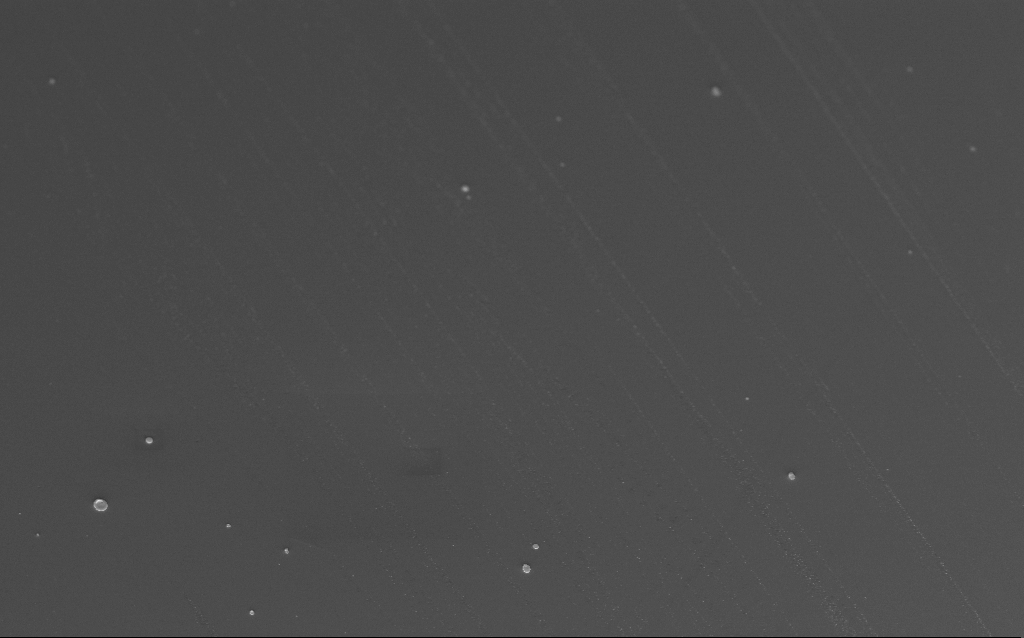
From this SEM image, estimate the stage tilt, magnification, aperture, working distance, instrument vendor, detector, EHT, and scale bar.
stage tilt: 40°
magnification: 2.99 K X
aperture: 30 µm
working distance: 5 mm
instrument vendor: Zeiss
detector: InLens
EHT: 3 kV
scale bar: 10000 nm